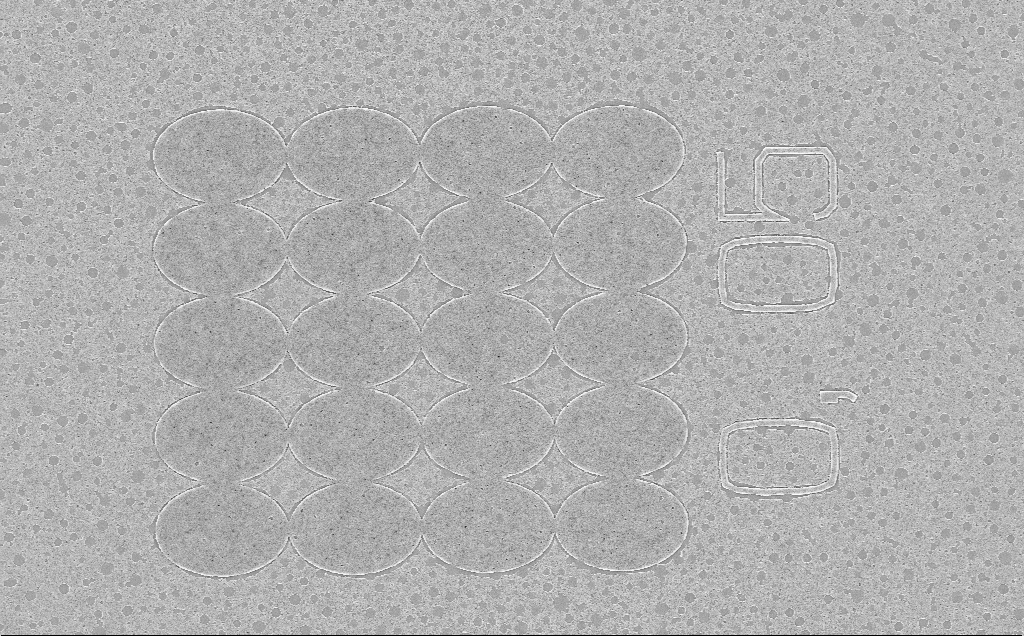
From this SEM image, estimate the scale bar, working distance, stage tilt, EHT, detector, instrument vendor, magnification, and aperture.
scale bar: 10000 nm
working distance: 6 mm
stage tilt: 0°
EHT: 5 kV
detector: InLens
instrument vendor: Zeiss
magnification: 4.92 K X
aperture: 30 µm